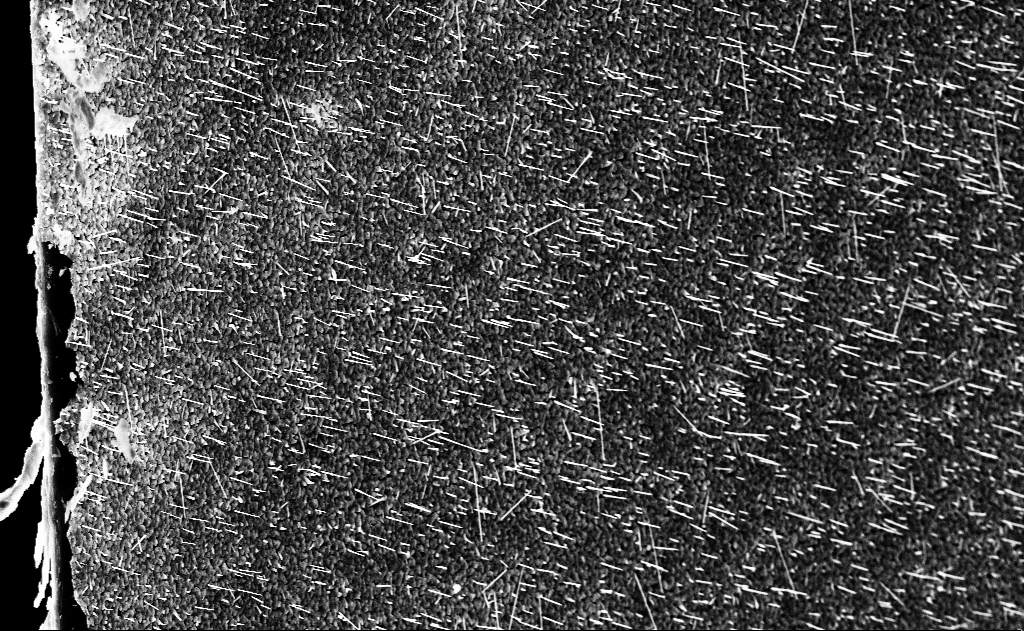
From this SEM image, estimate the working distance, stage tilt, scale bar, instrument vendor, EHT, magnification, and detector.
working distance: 14 mm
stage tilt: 0°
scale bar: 10000 nm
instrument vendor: Zeiss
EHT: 10 kV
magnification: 5 K X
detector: InLens